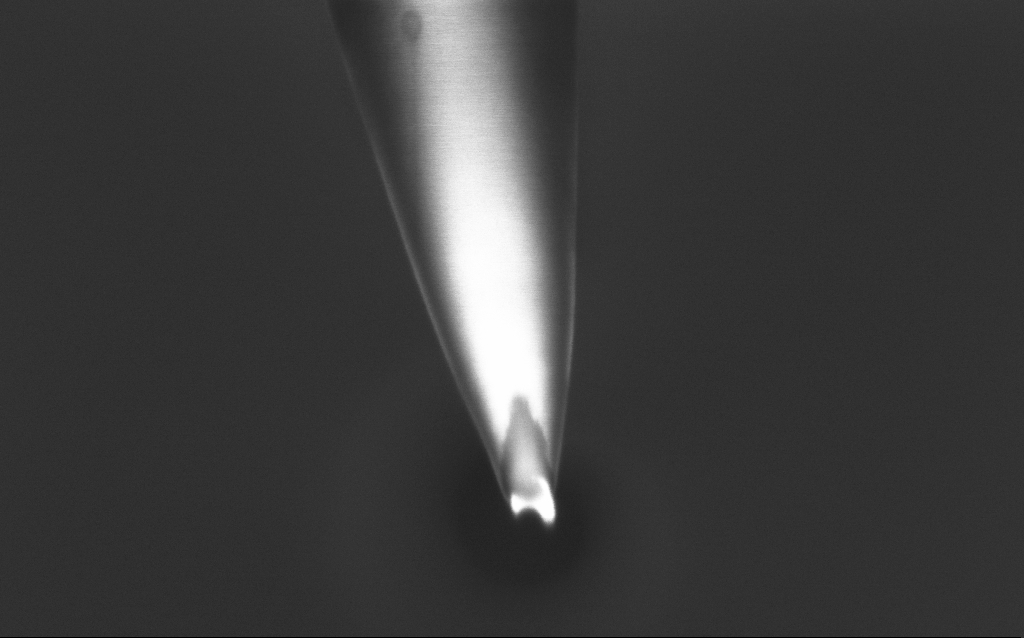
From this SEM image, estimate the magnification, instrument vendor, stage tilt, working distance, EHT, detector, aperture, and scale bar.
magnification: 99.65 K X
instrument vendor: Zeiss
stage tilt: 45°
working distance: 6 mm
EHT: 1 kV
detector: InLens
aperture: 30 µm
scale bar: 200 nm